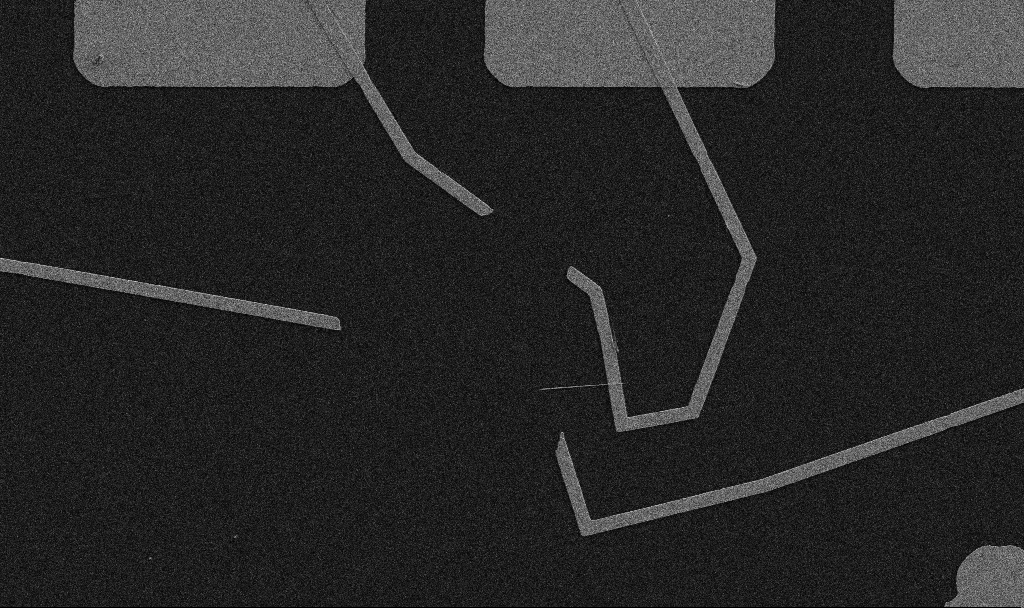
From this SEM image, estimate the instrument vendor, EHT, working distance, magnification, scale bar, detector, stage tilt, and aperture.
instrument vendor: Zeiss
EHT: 5 kV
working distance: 10.7 mm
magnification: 5 K X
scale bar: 10000 nm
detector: SE2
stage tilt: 0°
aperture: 30 µm